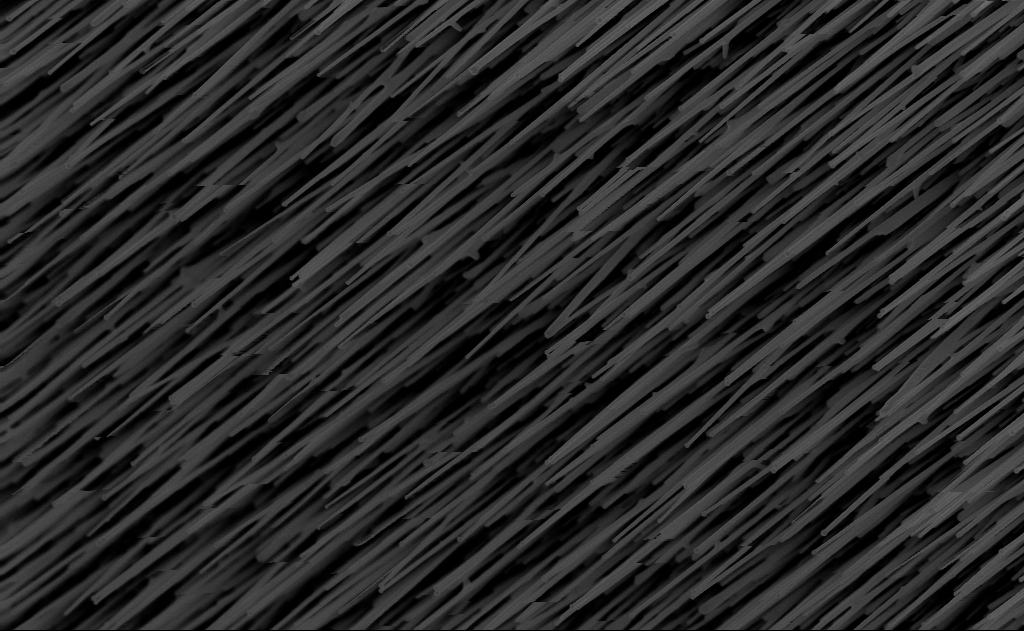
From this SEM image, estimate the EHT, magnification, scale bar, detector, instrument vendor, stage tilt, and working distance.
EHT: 10 kV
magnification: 20 K X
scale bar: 2000 nm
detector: InLens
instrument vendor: Zeiss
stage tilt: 0°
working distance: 10 mm